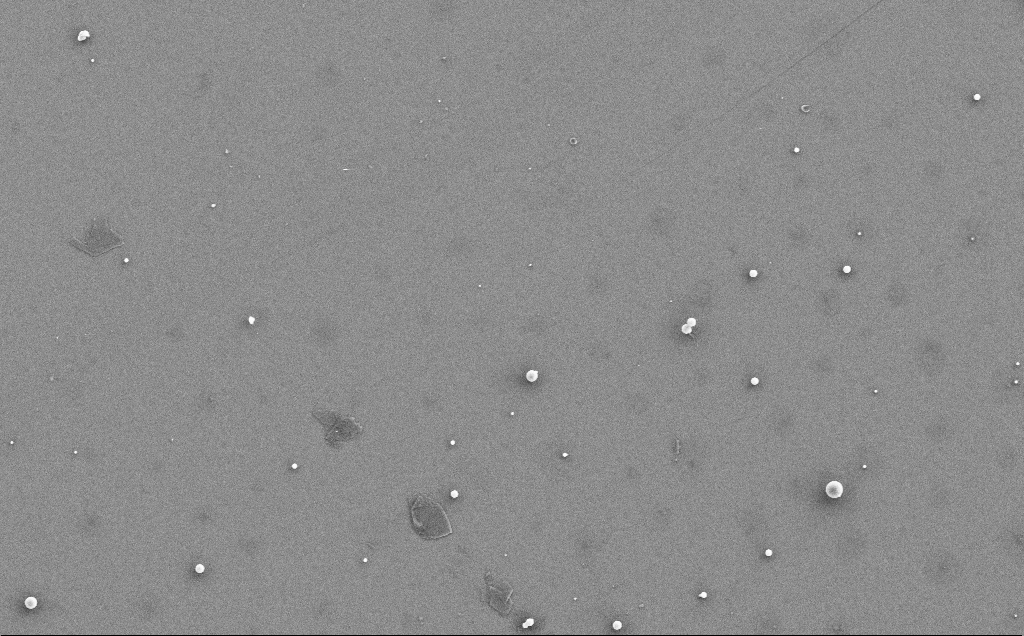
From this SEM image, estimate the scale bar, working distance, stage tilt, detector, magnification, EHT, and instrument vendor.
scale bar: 10000 nm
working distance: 5 mm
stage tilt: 0°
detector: SE2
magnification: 4.01 K X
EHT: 5 kV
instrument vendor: Zeiss